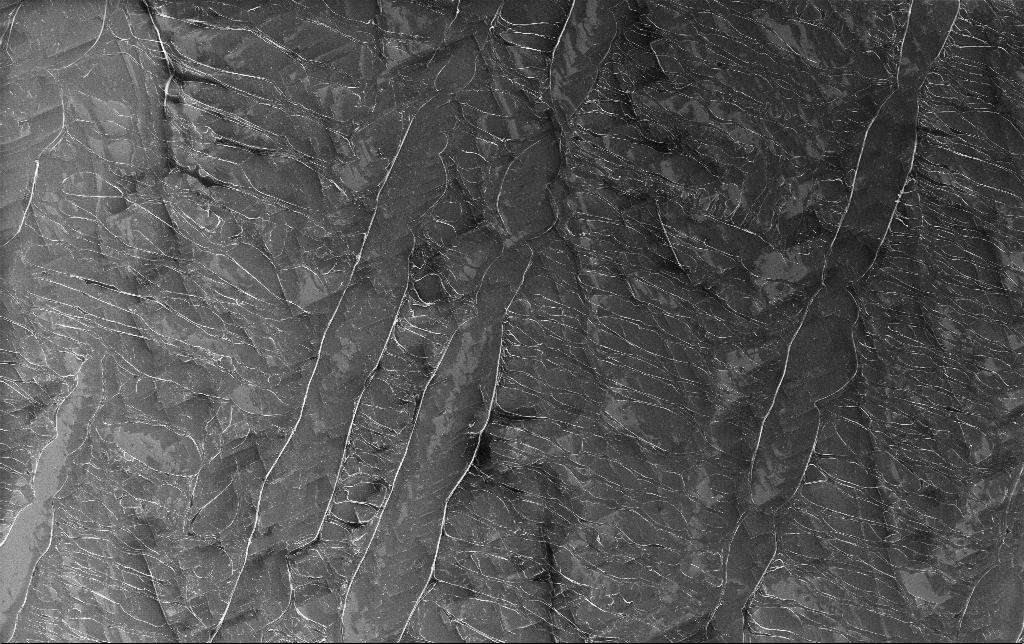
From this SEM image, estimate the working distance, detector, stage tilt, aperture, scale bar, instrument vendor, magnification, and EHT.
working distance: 3.1 mm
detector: InLens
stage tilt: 0°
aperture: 30 µm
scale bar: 20000 nm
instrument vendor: Zeiss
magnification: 0.972 K X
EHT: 5 kV